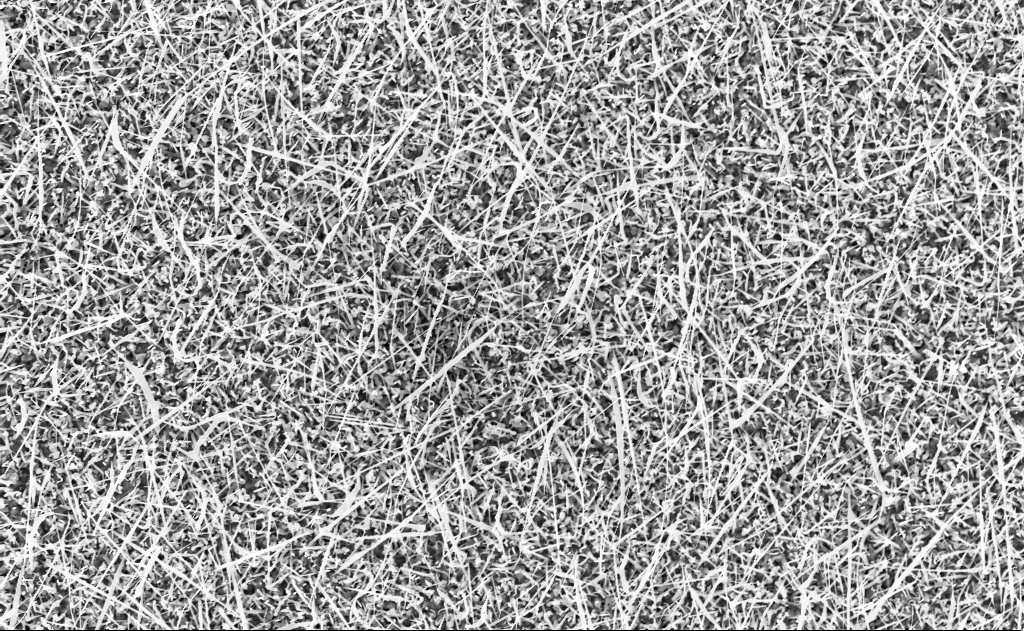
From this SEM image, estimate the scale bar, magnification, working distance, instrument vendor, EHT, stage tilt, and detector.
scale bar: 2000 nm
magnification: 10 K X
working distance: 10 mm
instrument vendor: Zeiss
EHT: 10 kV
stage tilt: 0°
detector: InLens